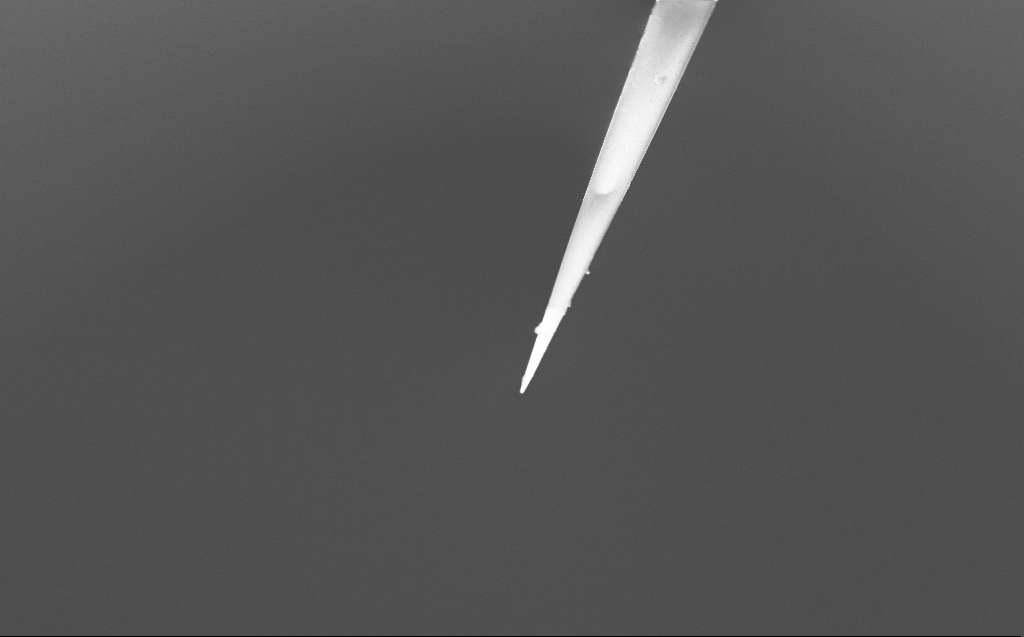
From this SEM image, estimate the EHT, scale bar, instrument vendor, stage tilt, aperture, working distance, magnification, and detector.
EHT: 2 kV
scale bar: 20000 nm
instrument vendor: Zeiss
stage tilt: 45°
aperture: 30 µm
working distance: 3 mm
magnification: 1 K X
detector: InLens